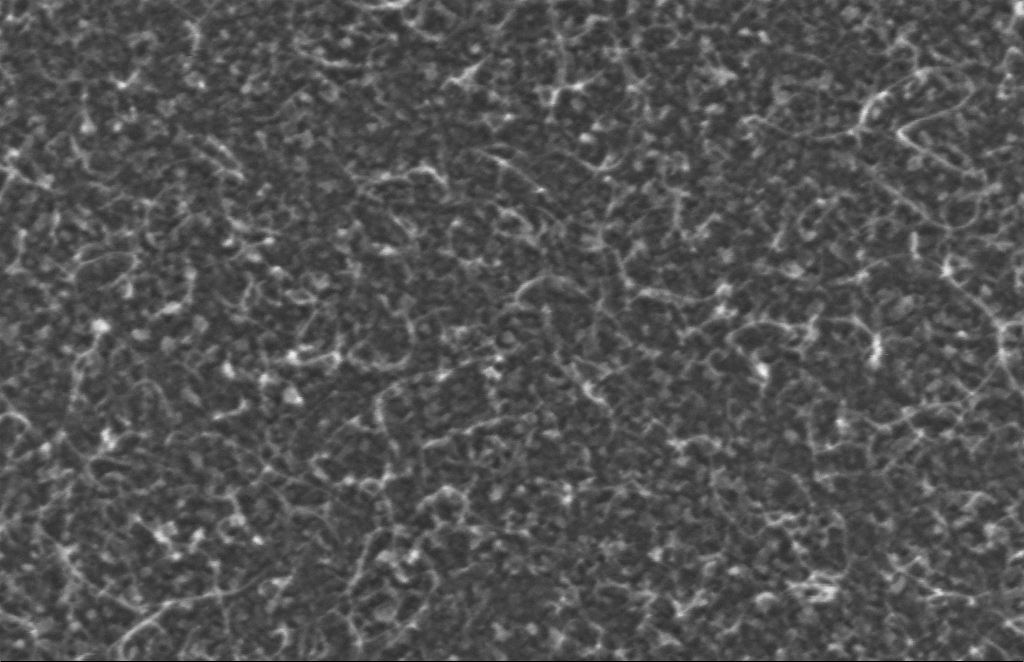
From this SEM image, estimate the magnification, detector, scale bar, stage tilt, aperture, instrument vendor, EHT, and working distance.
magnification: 393.75 K X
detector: InLens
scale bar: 100 nm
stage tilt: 0°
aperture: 30 µm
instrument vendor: Zeiss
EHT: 5 kV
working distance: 4 mm